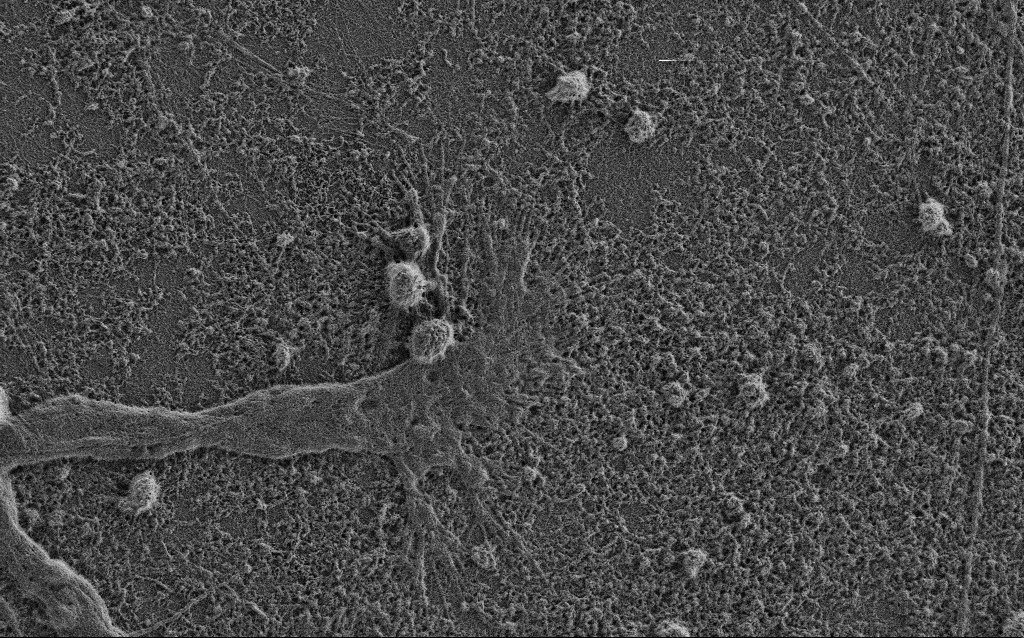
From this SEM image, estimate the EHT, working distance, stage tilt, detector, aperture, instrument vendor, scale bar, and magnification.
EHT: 0.9 kV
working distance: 3.4 mm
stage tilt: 0°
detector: SE2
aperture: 30 µm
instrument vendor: Zeiss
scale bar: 2000 nm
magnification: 8 K X